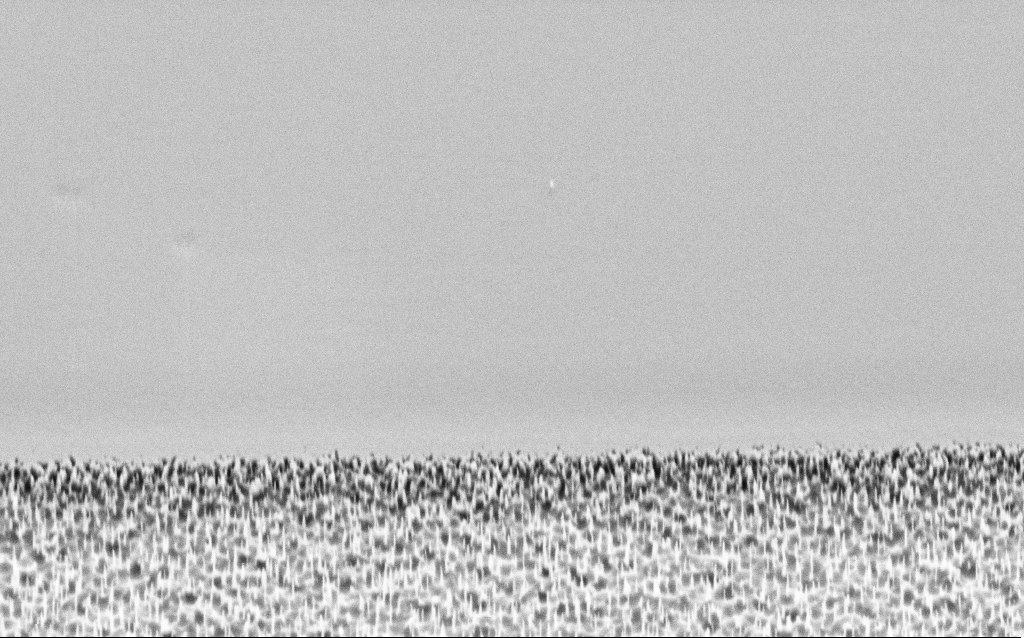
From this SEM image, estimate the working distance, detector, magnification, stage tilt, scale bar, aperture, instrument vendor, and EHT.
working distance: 8 mm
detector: SE2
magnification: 26.66 K X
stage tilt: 45°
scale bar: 2000 nm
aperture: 30 µm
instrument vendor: Zeiss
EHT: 3 kV